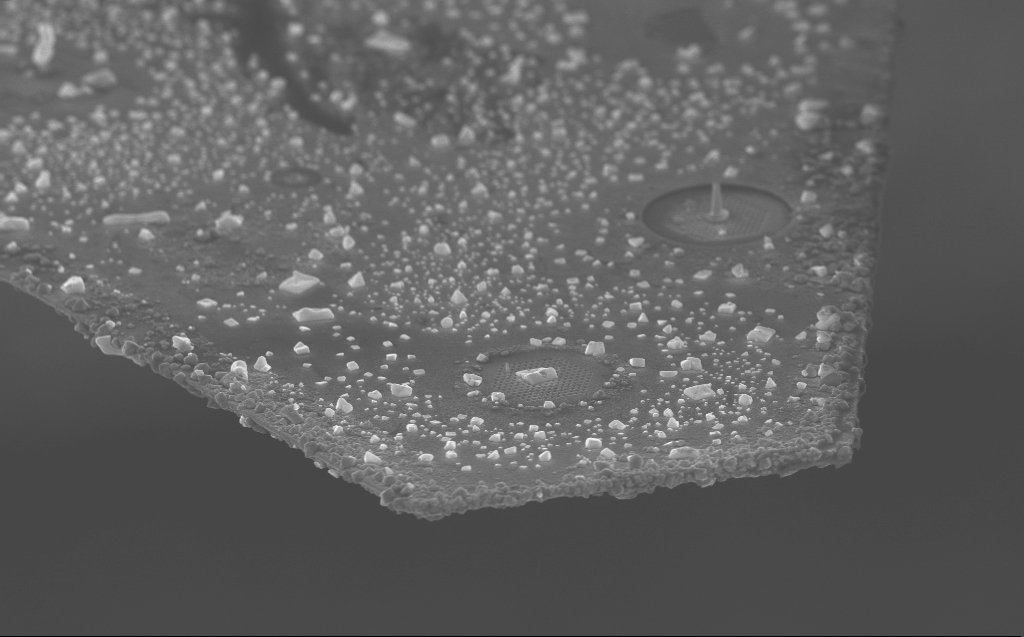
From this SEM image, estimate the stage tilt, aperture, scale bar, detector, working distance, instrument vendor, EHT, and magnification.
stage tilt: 60°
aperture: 30 µm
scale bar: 2000 nm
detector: InLens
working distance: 5 mm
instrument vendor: Zeiss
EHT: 10 kV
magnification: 9.32 K X